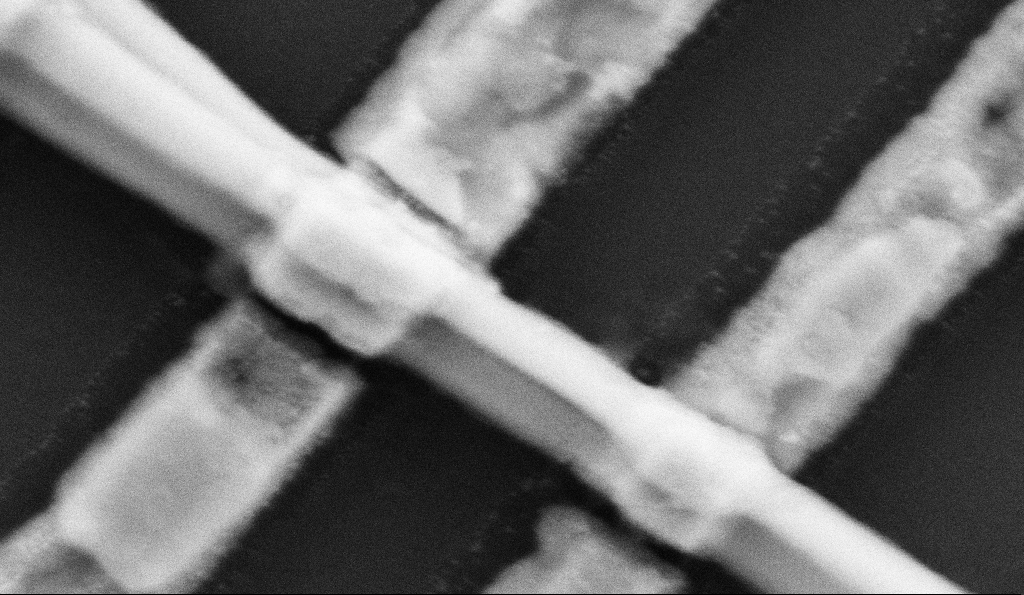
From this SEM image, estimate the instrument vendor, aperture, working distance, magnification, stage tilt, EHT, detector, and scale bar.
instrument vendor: Zeiss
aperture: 30 µm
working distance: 8.5 mm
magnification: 228.15 K X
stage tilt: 0°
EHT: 5 kV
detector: SE2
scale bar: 200 nm